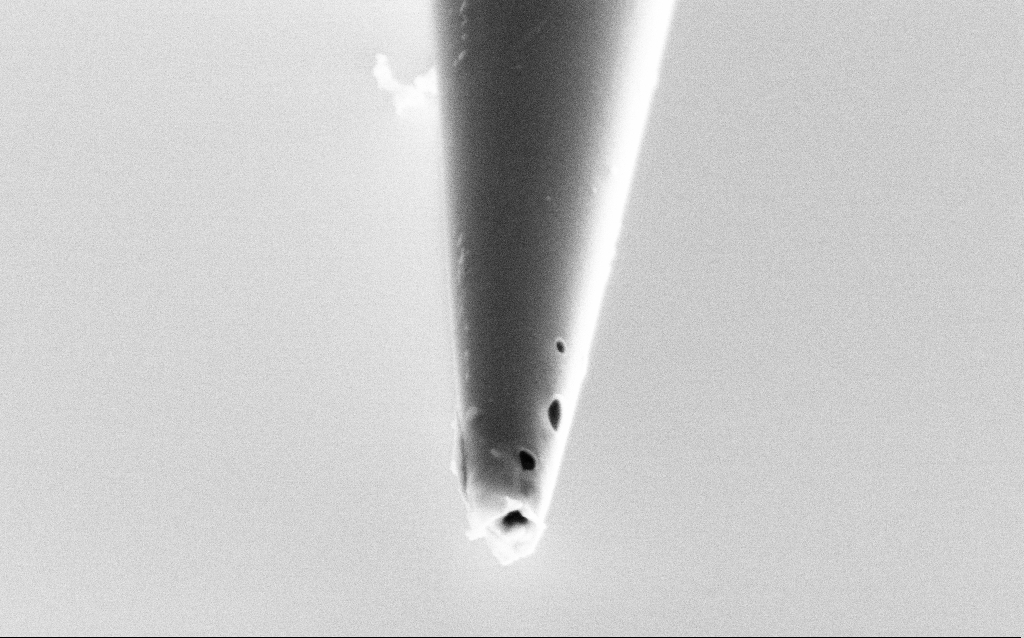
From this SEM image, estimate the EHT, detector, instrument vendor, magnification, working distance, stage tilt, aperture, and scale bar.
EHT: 2 kV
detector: SE2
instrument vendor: Zeiss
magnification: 100 K X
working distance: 6 mm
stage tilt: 45°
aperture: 30 µm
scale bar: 200 nm